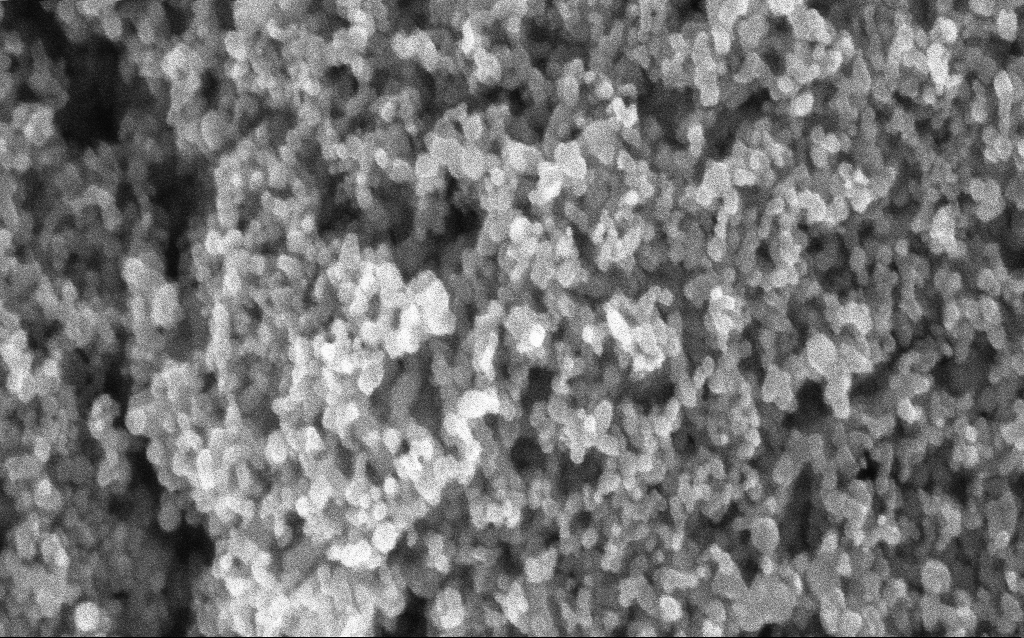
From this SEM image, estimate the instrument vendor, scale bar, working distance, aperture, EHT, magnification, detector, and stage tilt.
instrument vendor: Zeiss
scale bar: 100 nm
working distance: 2.7 mm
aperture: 30 µm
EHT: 5 kV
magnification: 211.33 K X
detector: InLens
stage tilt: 0°